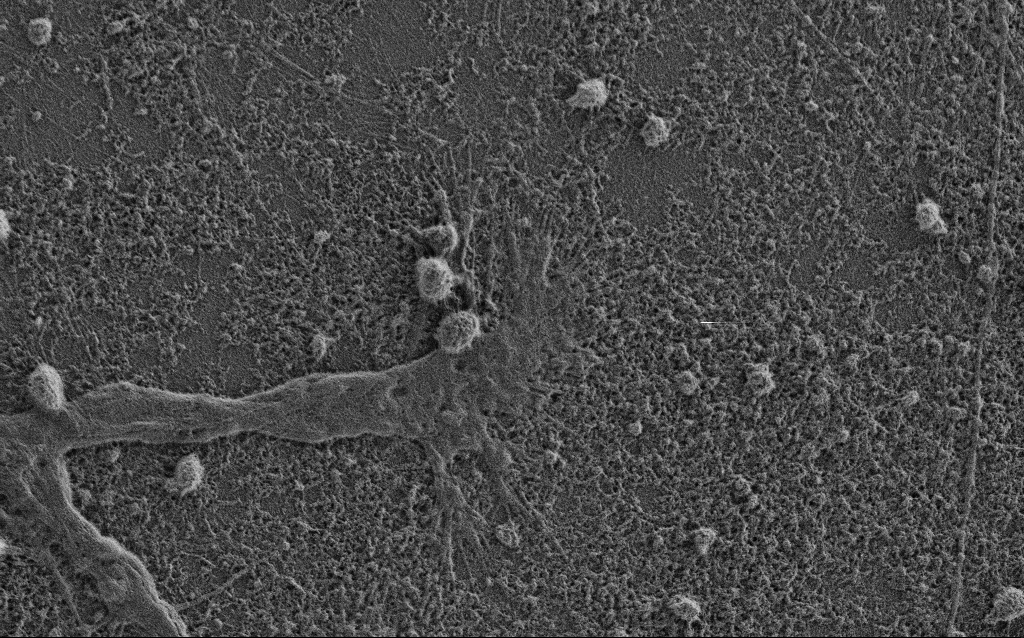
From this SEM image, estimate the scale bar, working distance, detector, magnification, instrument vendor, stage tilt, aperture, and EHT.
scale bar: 2000 nm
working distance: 3.4 mm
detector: SE2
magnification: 7.5 K X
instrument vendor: Zeiss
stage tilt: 0°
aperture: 30 µm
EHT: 0.9 kV